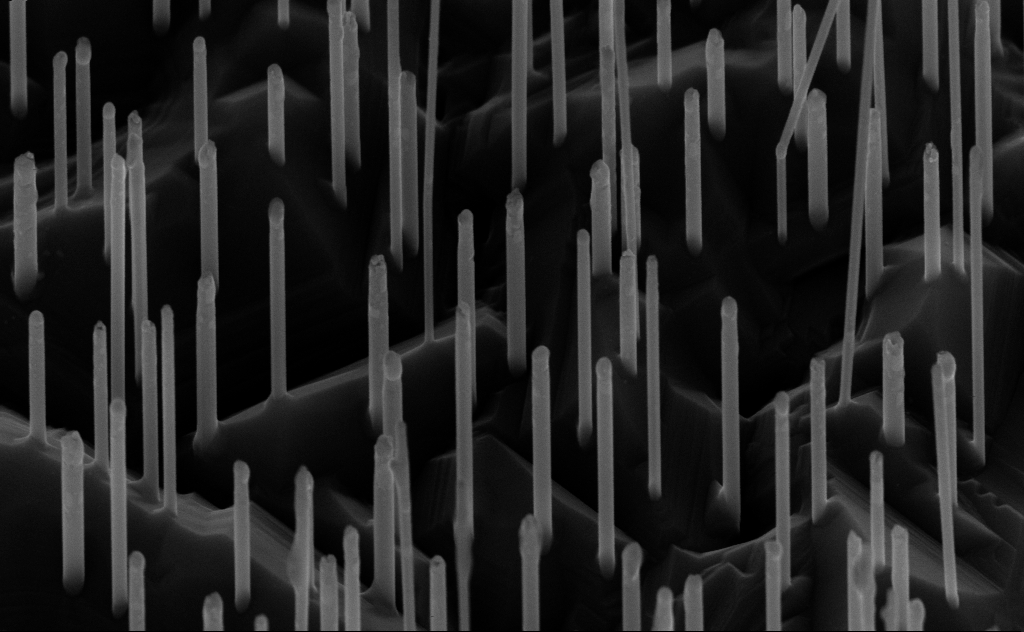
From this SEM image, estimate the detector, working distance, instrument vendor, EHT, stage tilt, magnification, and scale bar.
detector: InLens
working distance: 6 mm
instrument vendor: Zeiss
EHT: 10 kV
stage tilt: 45°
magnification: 80 K X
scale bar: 200 nm